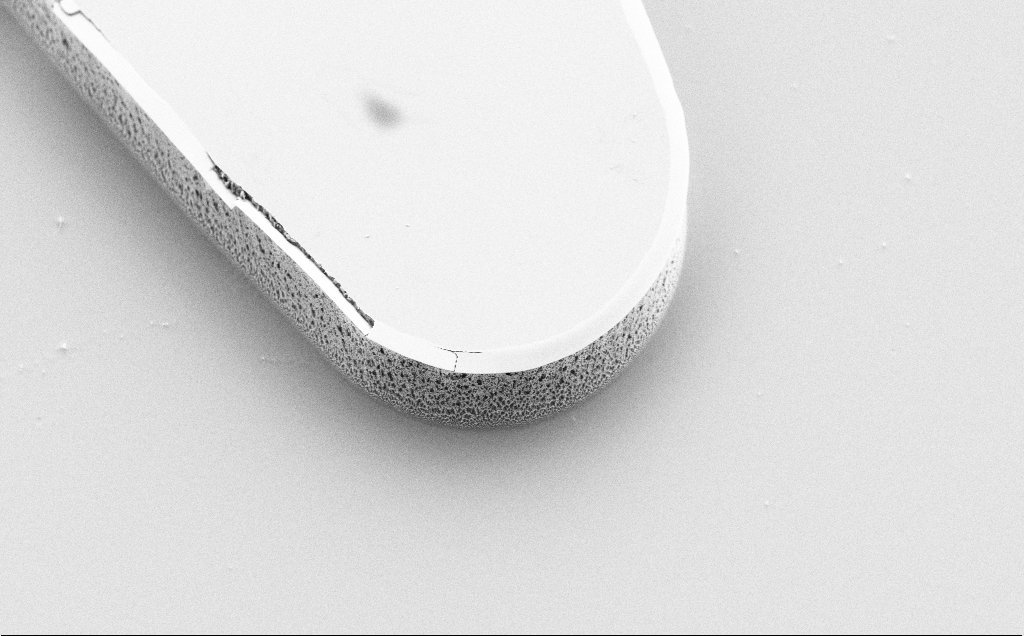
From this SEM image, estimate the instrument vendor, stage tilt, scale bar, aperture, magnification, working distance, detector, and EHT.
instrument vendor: Zeiss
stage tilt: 45°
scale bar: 20000 nm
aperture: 30 µm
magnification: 1.88 K X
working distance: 8 mm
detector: SE2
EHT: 5 kV